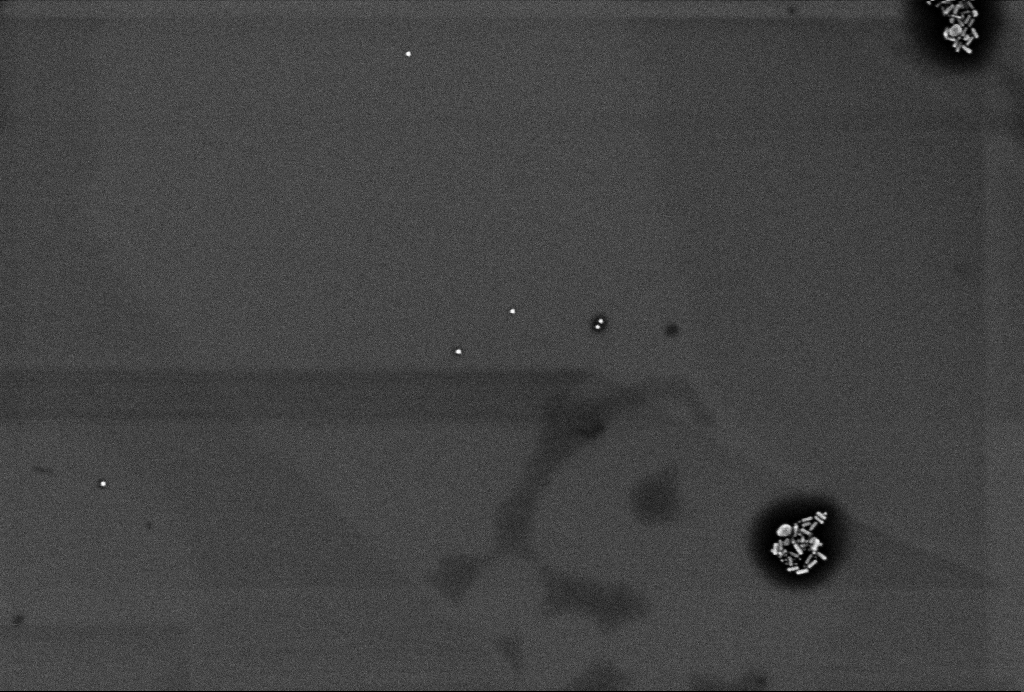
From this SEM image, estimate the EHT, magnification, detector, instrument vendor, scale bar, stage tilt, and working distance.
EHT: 2 kV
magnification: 60.84 K X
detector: InLens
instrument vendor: Zeiss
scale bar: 200 nm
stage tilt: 0°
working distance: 3.3 mm